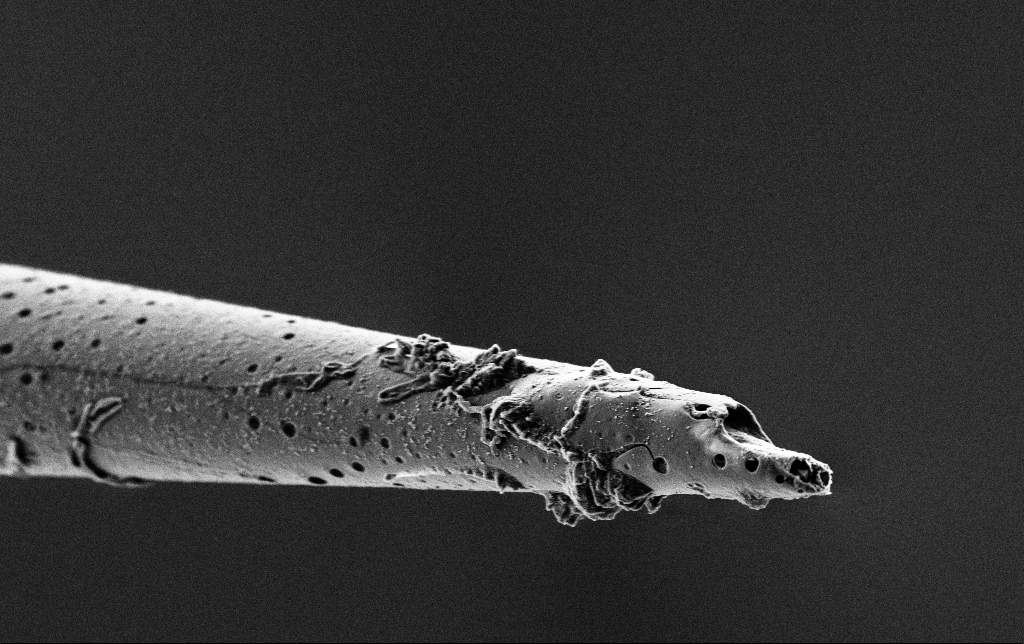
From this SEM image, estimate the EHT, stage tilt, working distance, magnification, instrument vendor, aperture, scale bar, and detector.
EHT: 2 kV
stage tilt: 45°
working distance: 7.2 mm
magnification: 10 K X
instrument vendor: Zeiss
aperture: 30 µm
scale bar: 2000 nm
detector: SE2